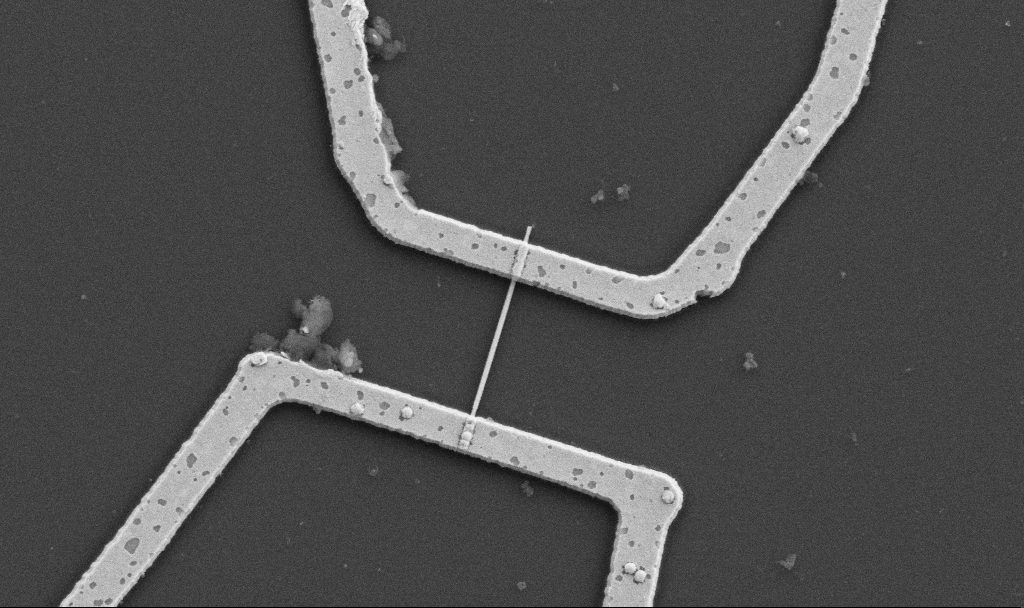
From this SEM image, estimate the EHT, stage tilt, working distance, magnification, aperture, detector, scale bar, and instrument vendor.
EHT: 5 kV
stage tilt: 0°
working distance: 10.7 mm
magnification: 20 K X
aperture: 30 µm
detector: SE2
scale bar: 1000 nm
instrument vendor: Zeiss